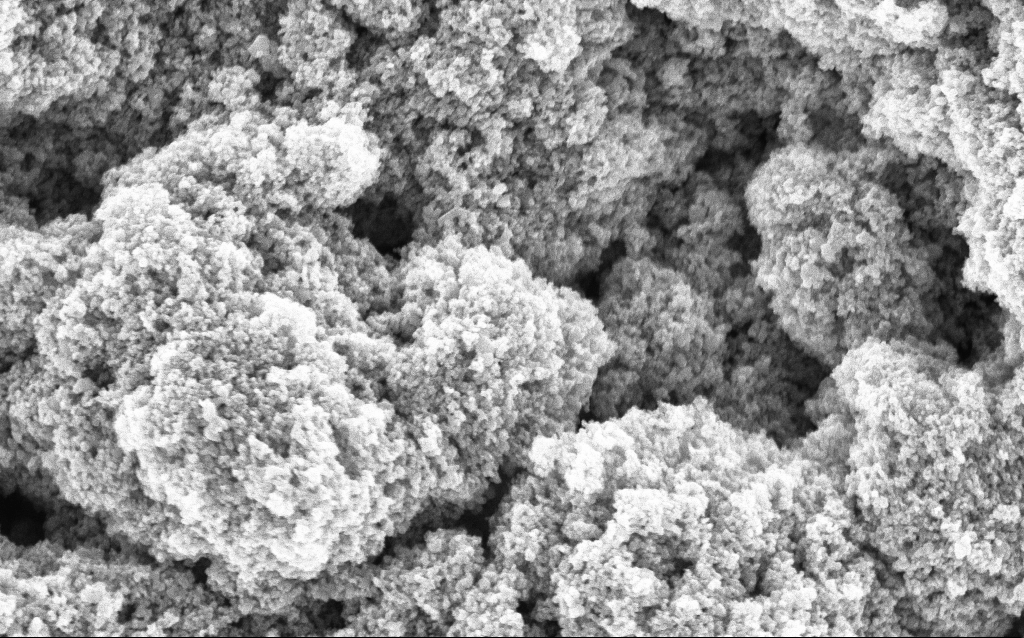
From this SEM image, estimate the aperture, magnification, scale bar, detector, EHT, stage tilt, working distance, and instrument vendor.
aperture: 30 µm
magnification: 68.7 K X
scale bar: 1000 nm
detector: InLens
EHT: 5 kV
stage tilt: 0°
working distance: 4.6 mm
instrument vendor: Zeiss